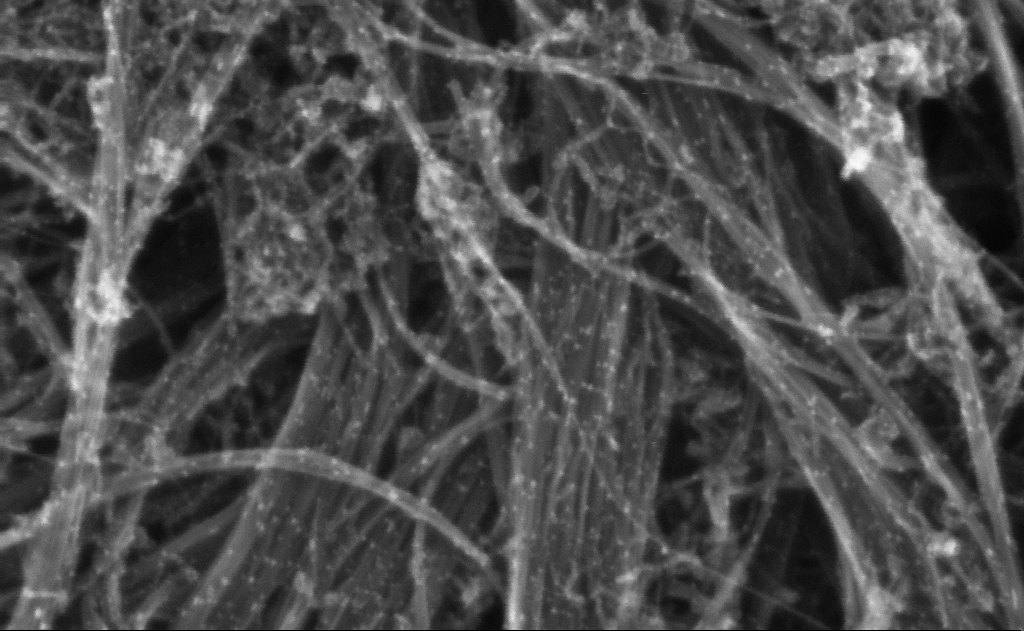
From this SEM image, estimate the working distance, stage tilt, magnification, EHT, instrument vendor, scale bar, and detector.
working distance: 3 mm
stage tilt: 0°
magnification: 566.11 K X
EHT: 10 kV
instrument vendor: Zeiss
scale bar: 100 nm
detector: InLens